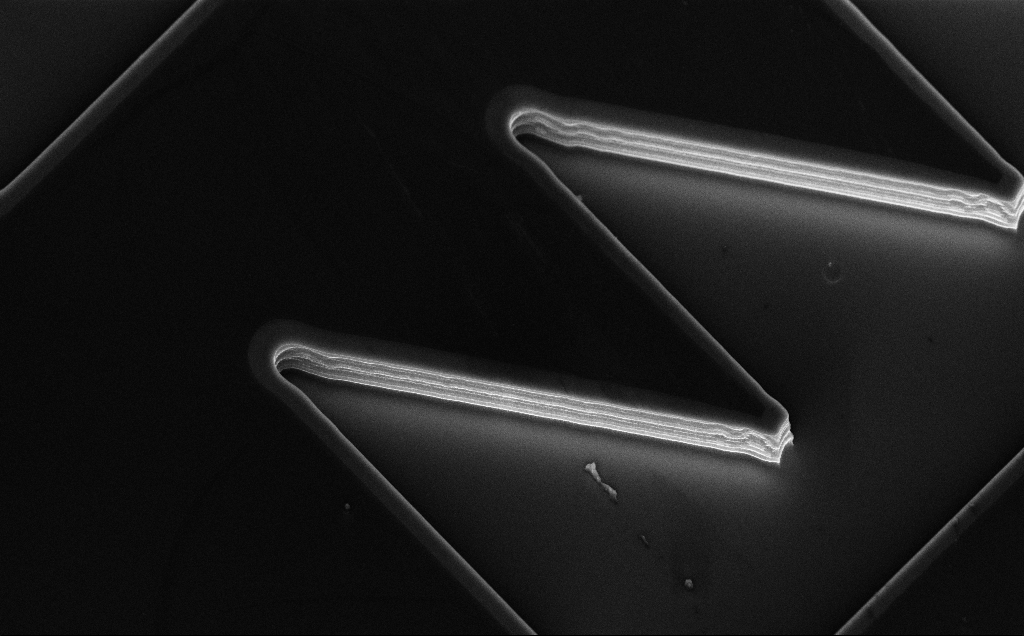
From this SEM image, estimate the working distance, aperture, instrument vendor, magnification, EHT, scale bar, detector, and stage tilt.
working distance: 10 mm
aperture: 30 µm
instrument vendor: Zeiss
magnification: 3.8 K X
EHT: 10 kV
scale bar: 10000 nm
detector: InLens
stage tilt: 50°